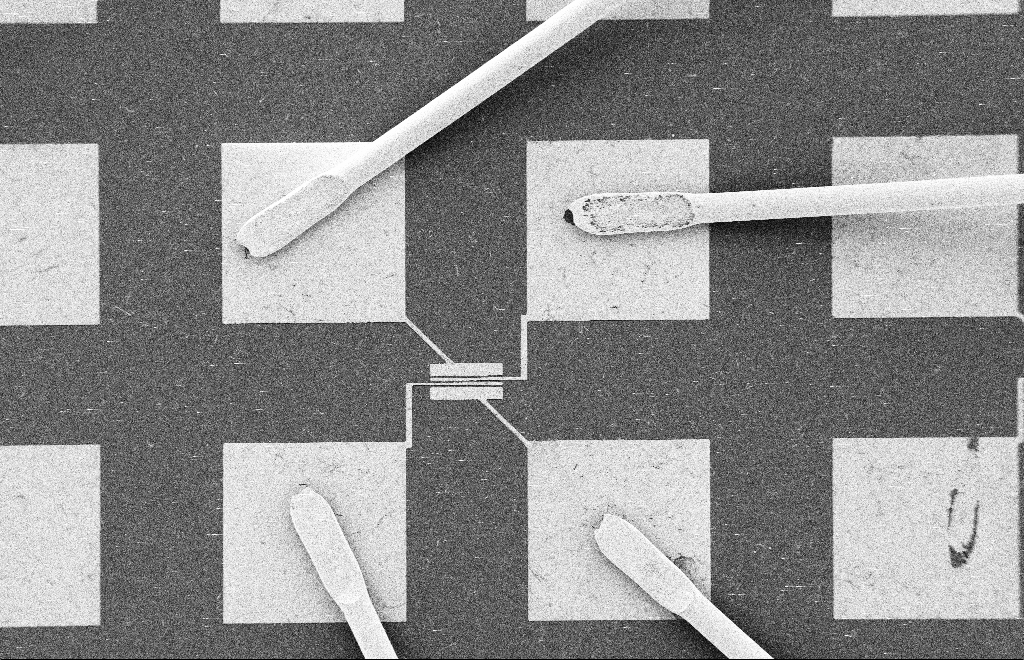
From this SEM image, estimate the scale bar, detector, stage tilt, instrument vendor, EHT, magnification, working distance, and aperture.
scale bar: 100000 nm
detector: SE2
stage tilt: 0°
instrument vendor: Zeiss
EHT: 2 kV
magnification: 0.442 K X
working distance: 10 mm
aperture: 20 µm